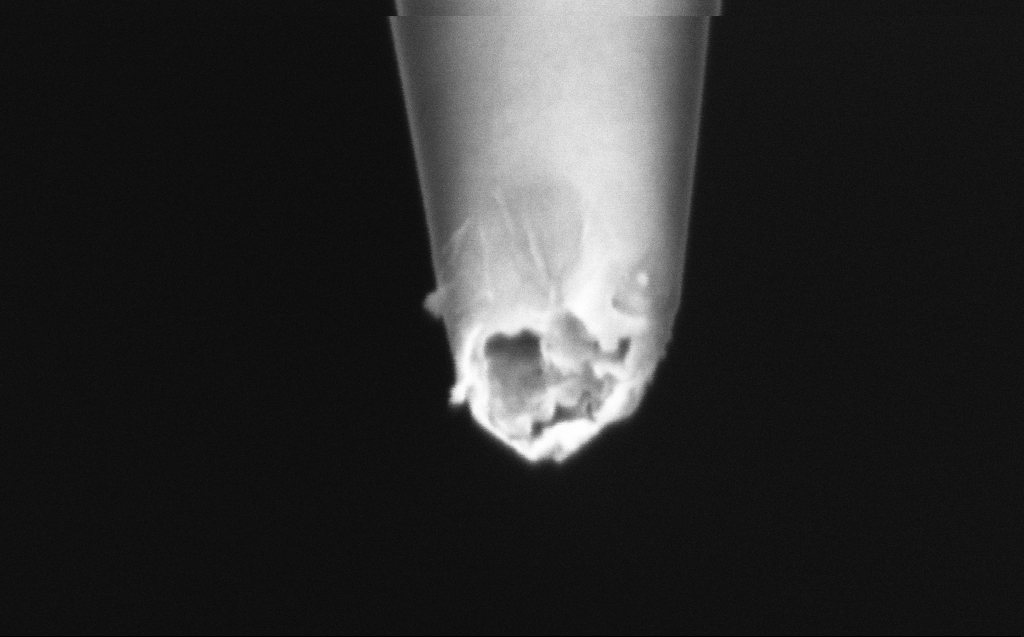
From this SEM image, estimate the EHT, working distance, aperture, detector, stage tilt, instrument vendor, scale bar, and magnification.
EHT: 2 kV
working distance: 6 mm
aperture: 30 µm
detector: InLens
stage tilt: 45°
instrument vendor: Zeiss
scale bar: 200 nm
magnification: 250 K X